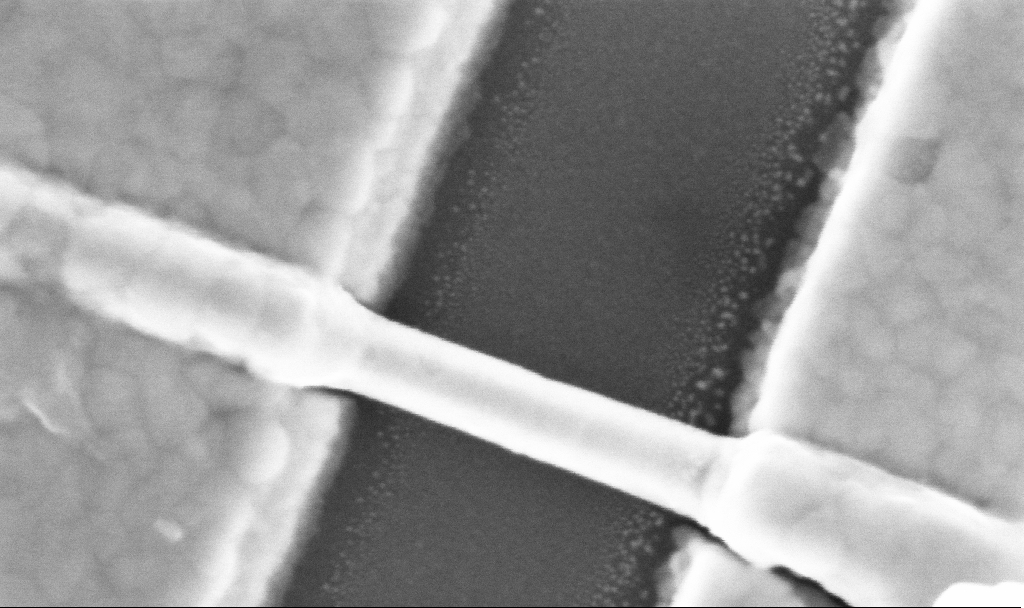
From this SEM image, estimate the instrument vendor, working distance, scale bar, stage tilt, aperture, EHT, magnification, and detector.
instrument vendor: Zeiss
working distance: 6.7 mm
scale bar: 200 nm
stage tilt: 0°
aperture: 30 µm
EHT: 5 kV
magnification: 300 K X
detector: InLens